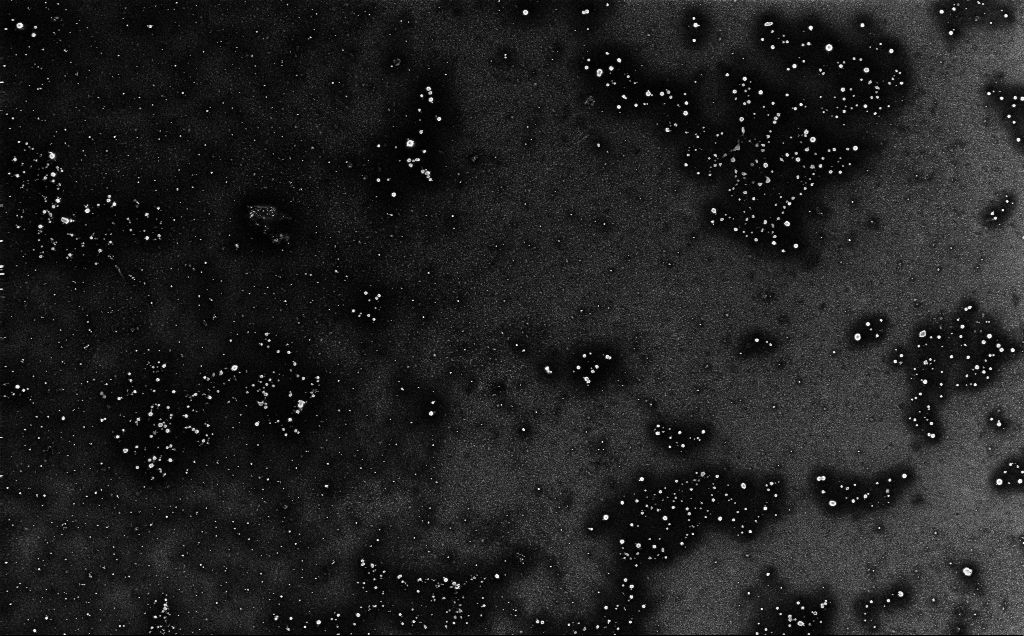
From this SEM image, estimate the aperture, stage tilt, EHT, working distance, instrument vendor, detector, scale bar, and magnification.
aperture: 30 µm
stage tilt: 0°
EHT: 10 kV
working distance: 3.2 mm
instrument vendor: Zeiss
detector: InLens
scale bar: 10000 nm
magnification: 1.43 K X